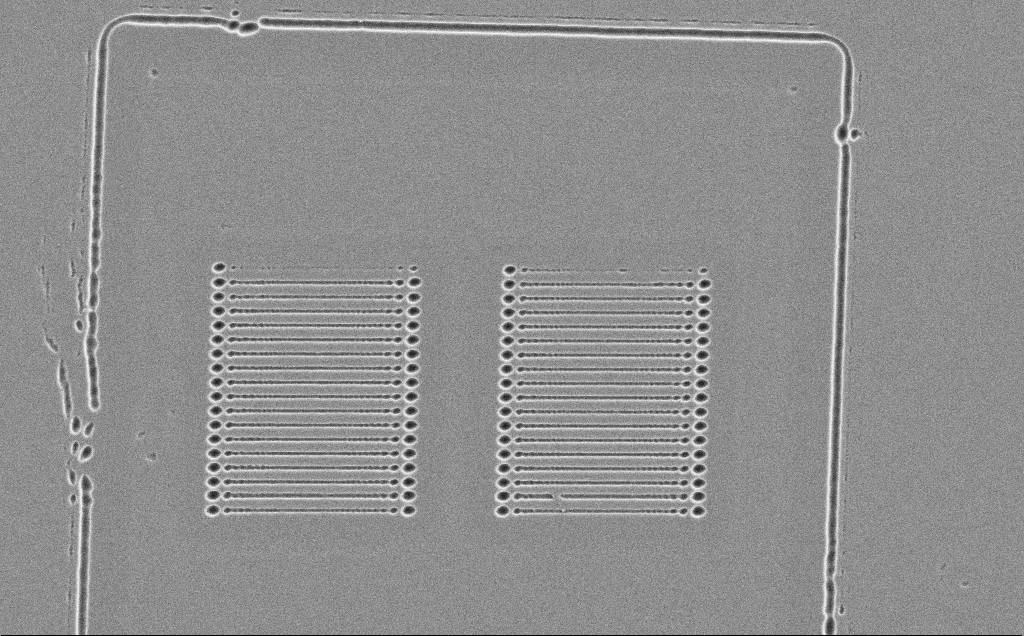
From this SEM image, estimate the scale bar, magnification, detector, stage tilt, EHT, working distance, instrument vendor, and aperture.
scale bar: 20000 nm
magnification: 2.65 K X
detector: SE2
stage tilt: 0°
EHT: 5 kV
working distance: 10 mm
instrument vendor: Zeiss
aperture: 30 µm